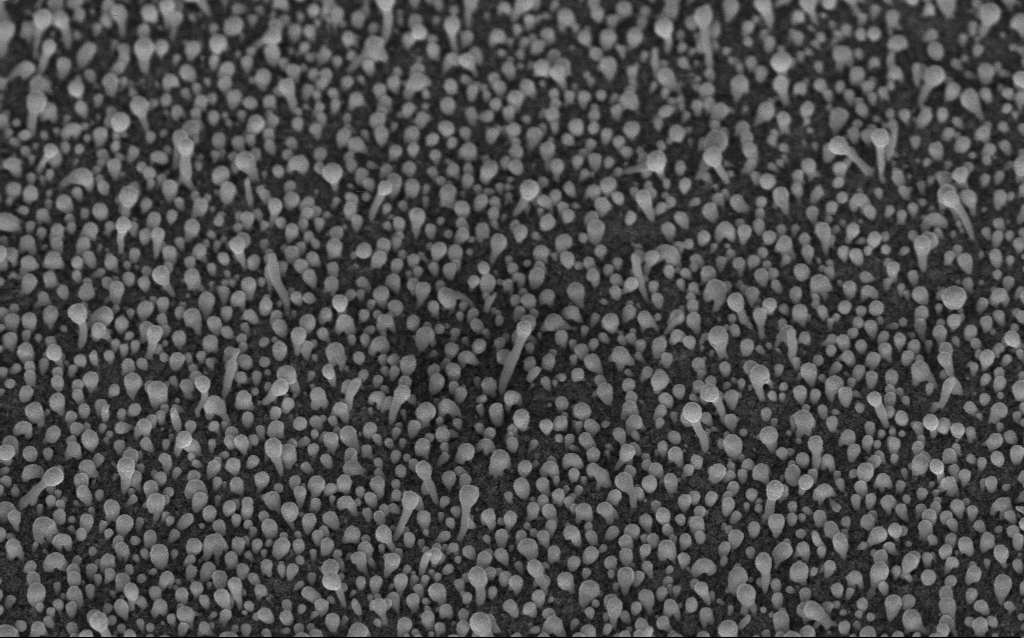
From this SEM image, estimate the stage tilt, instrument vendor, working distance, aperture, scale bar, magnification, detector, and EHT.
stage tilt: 45°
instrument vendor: Zeiss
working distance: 6.1 mm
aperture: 30 µm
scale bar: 1000 nm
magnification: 50 K X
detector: InLens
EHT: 5 kV